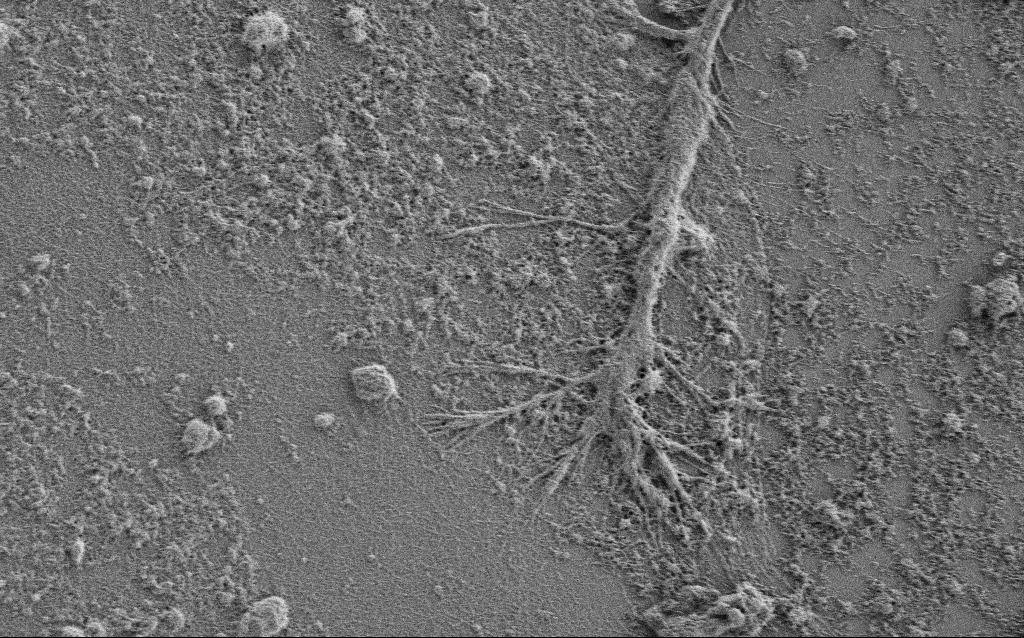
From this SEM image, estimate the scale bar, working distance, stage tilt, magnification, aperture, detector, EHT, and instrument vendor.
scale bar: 10000 nm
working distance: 4 mm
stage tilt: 0°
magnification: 5 K X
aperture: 30 µm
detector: SE2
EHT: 0.9 kV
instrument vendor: Zeiss